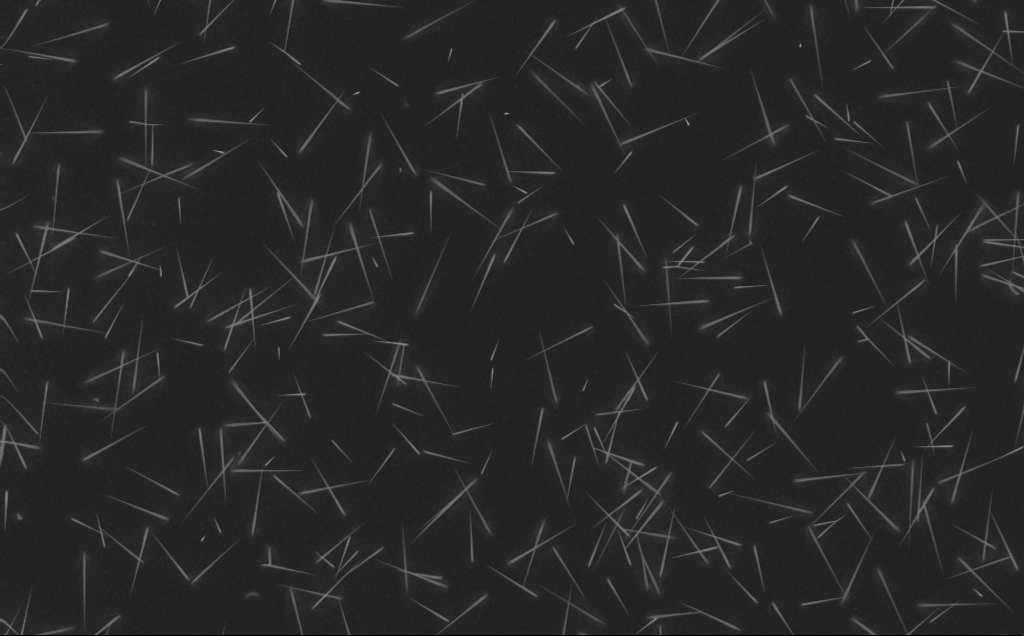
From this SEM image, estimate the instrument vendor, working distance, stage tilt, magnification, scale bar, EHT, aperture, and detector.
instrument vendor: Zeiss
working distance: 5 mm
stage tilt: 0°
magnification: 5 K X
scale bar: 10000 nm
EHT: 10 kV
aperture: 30 µm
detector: InLens